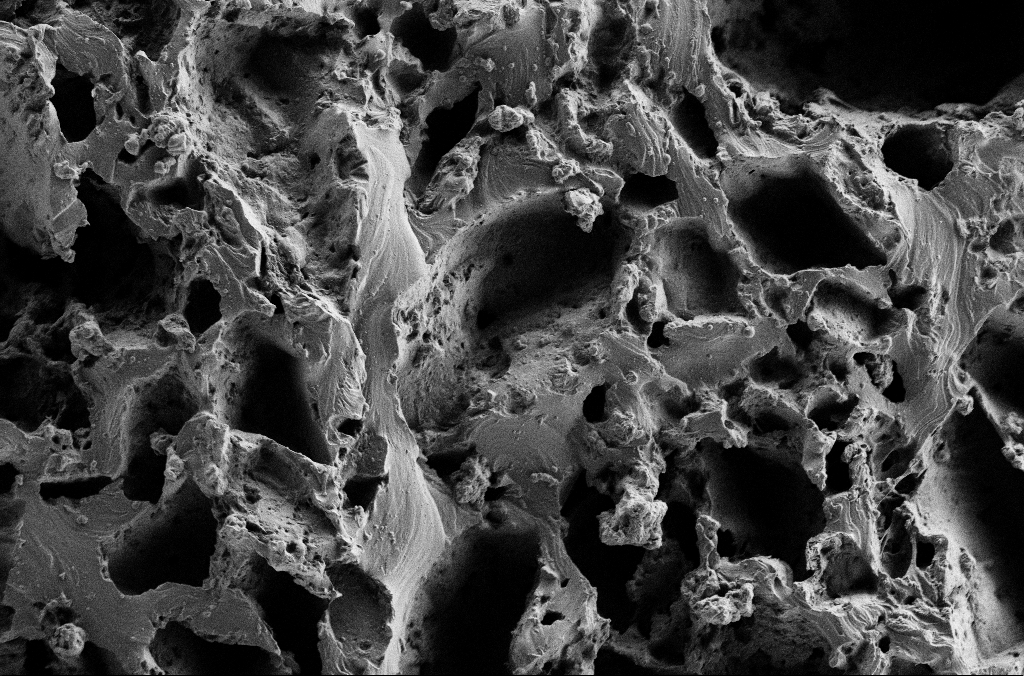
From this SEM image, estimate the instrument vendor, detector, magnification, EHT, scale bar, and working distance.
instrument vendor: Zeiss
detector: SE2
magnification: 1 K X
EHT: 2 kV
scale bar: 20000 nm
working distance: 3 mm